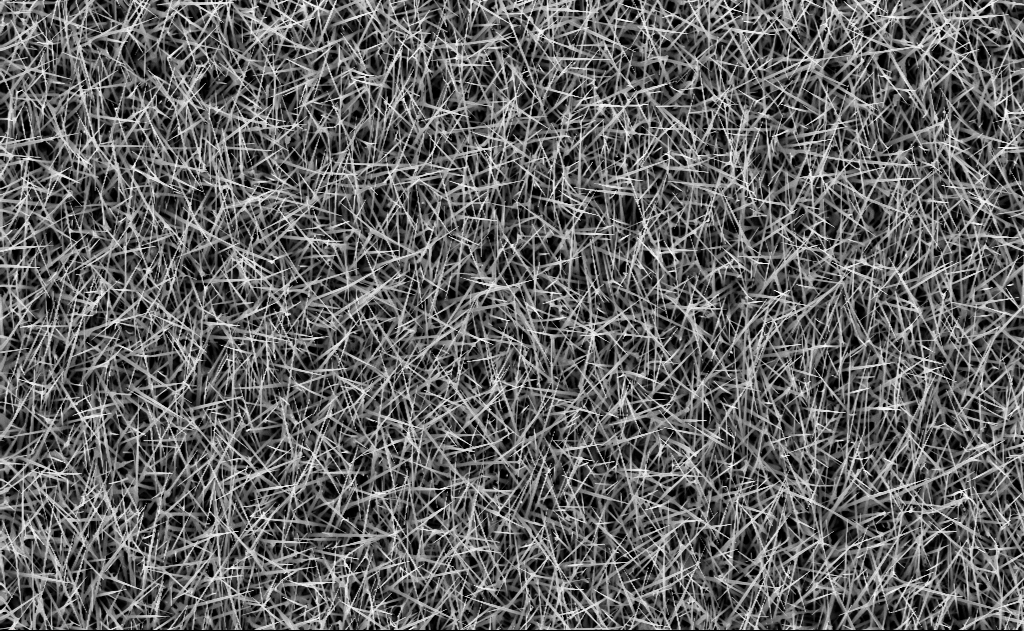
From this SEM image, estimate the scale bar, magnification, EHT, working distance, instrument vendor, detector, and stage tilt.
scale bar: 2000 nm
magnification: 10 K X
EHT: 10 kV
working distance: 7 mm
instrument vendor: Zeiss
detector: InLens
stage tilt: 0°